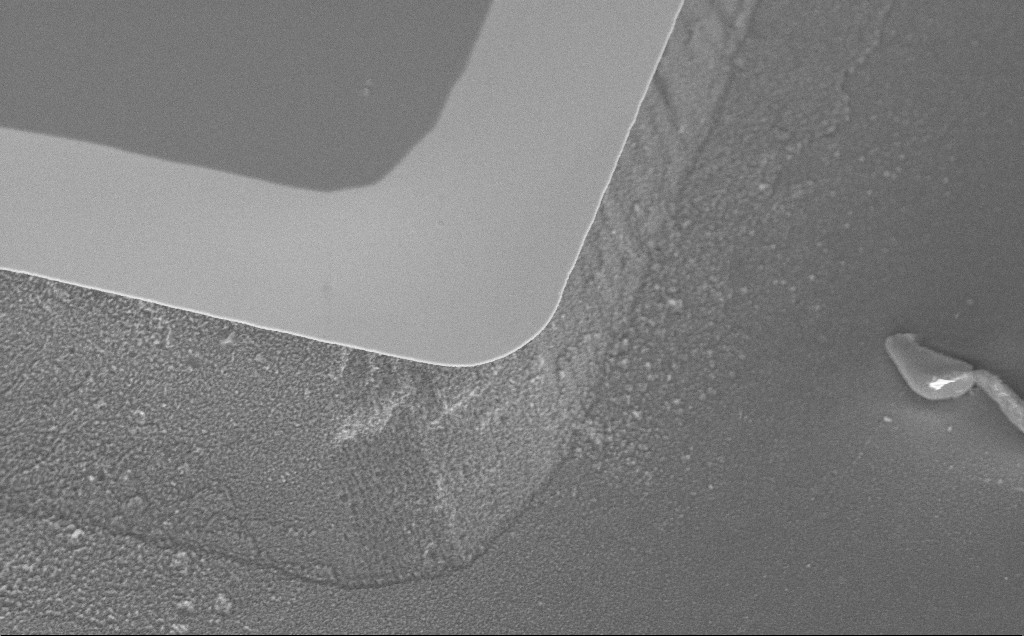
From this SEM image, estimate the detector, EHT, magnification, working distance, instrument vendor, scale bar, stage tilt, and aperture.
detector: InLens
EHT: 5 kV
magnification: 9.09 K X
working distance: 13 mm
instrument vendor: Zeiss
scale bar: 2000 nm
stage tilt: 45°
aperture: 30 µm